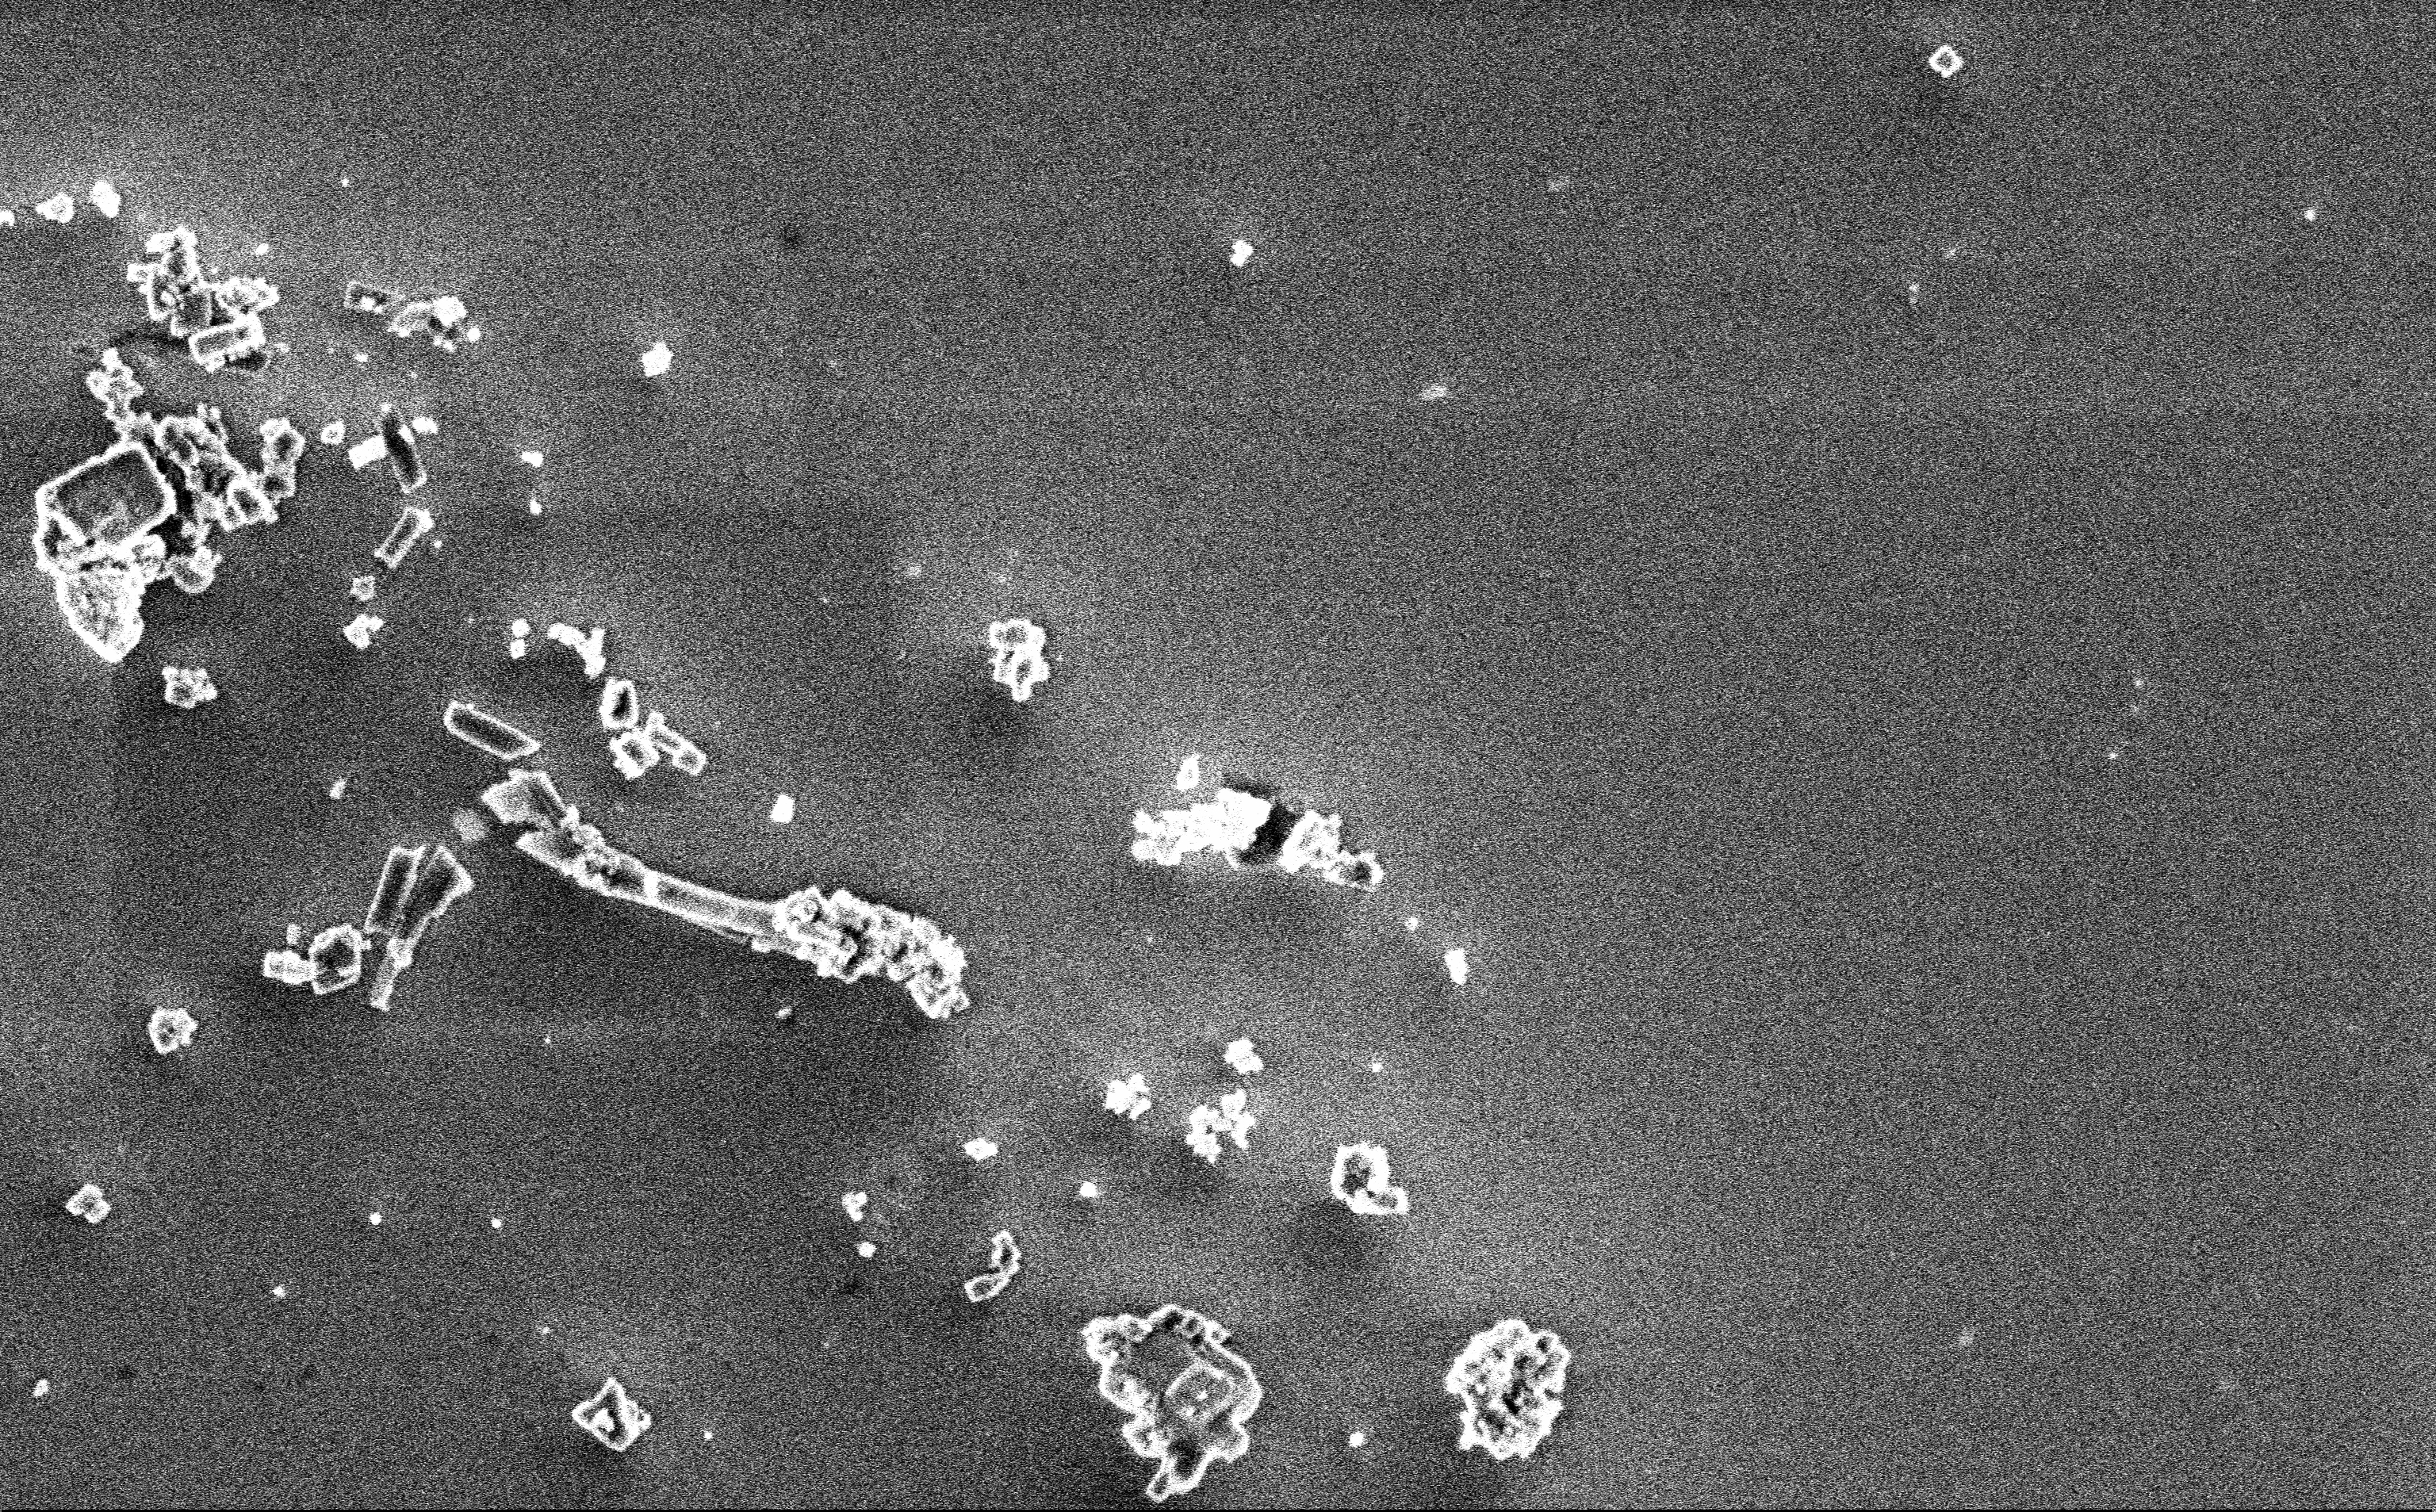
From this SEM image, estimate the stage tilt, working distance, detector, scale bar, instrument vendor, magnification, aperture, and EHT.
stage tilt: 0°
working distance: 3 mm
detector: InLens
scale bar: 2000 nm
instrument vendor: Zeiss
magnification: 12.85 K X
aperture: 30 µm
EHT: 3 kV